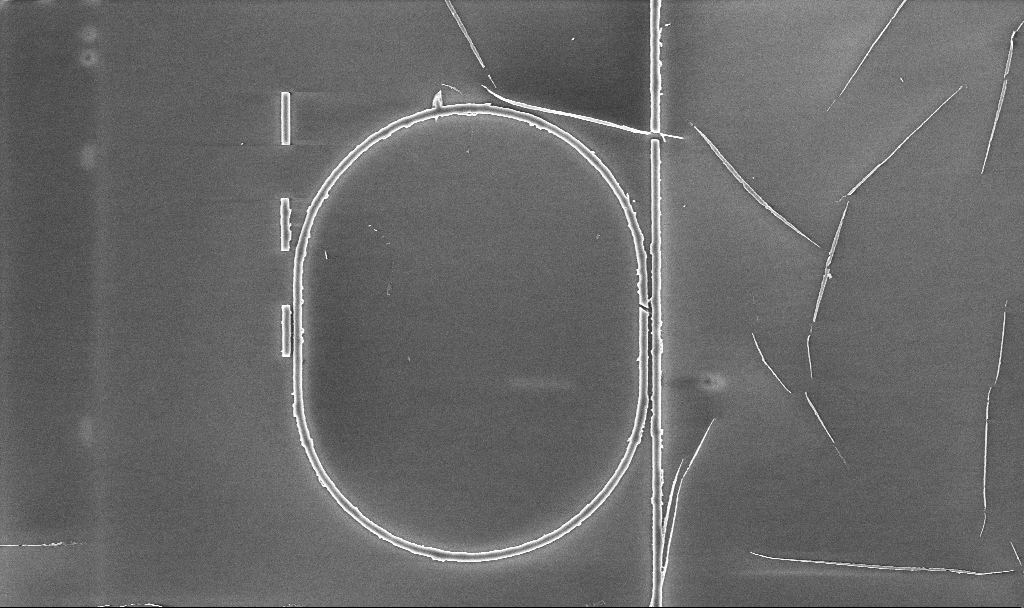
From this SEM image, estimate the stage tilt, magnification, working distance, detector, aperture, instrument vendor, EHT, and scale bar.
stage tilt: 0°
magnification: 6.58 K X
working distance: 5.2 mm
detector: InLens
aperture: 30 µm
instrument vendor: Zeiss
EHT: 5 kV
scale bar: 10000 nm